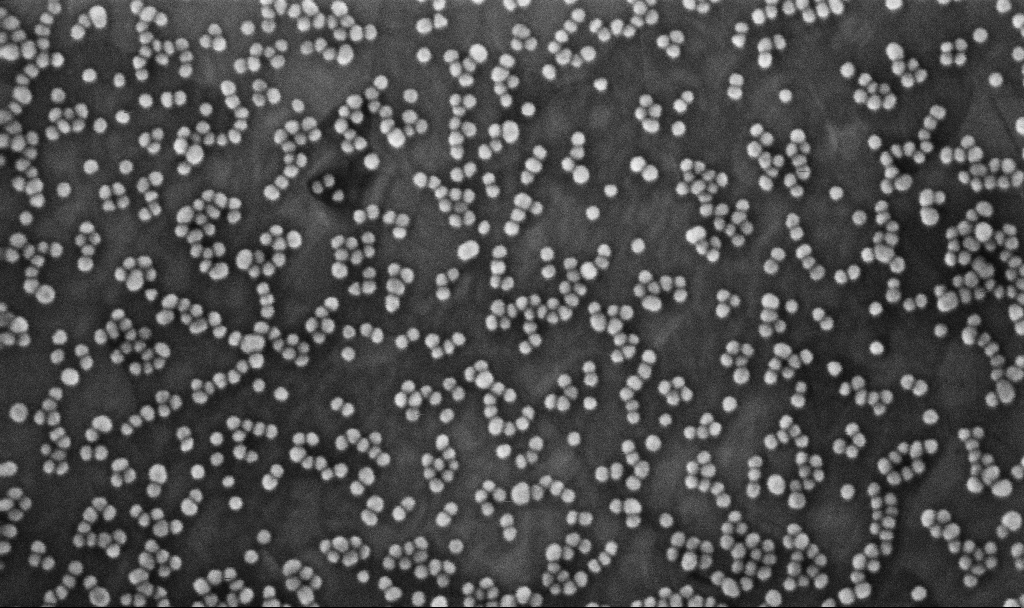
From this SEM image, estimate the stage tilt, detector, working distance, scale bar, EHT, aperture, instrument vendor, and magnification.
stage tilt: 0°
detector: InLens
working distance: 3.3 mm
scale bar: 100 nm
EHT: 10 kV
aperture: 30 µm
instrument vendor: Zeiss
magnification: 300 K X